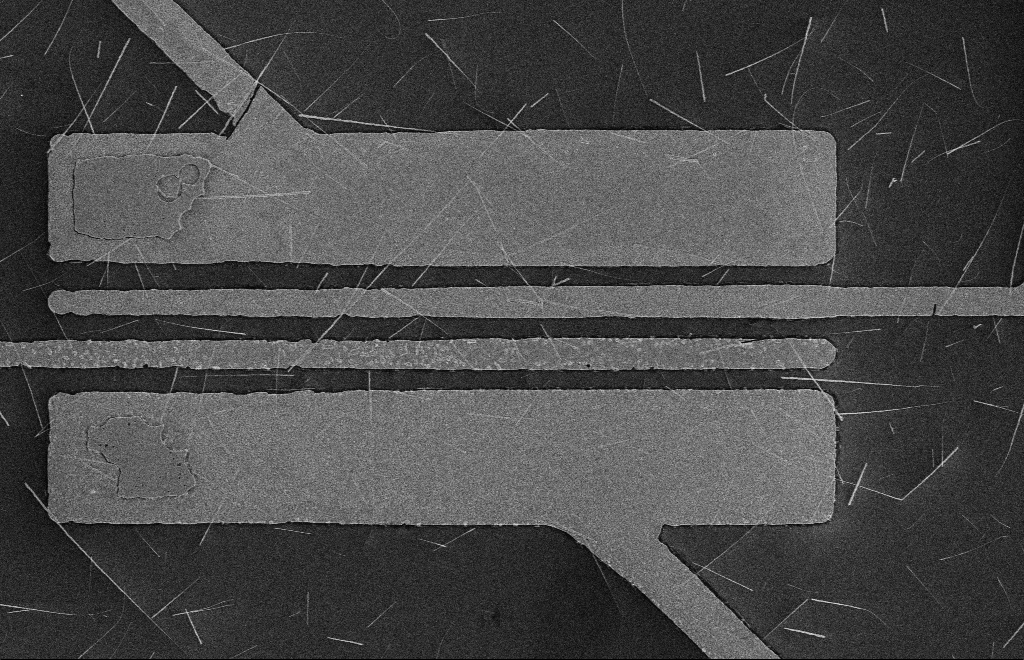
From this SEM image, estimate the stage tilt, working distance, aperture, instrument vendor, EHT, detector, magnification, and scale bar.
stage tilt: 0°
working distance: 16 mm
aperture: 10 µm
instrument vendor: Zeiss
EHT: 5 kV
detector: SE2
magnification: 4.79 K X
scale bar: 2000 nm